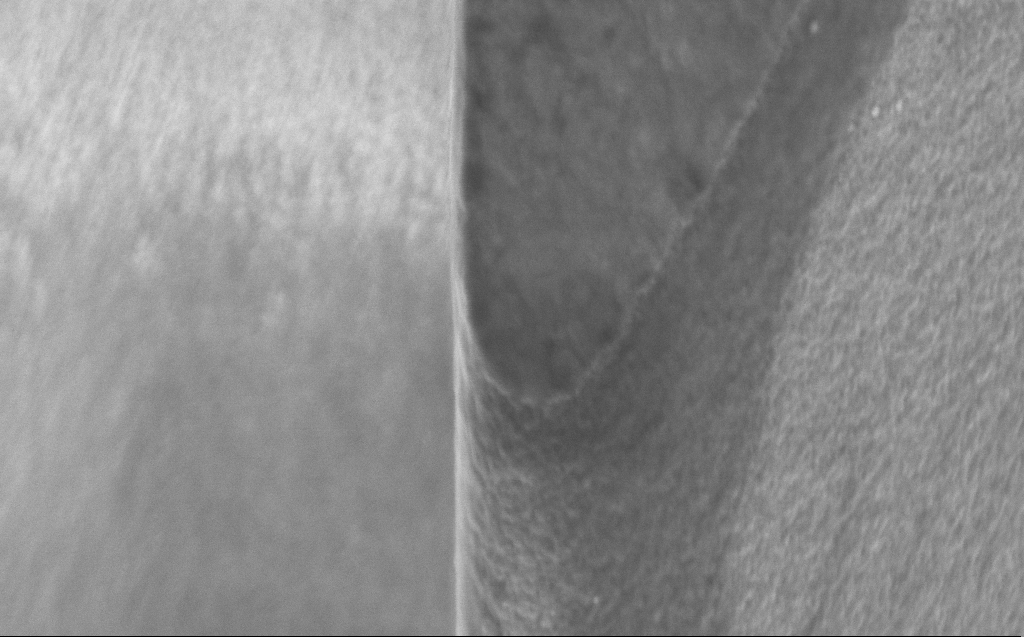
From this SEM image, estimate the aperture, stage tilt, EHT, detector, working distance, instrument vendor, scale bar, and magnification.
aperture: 30 µm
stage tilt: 45.1°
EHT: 3 kV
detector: InLens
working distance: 8 mm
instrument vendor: Zeiss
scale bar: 1000 nm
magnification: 69.9 K X